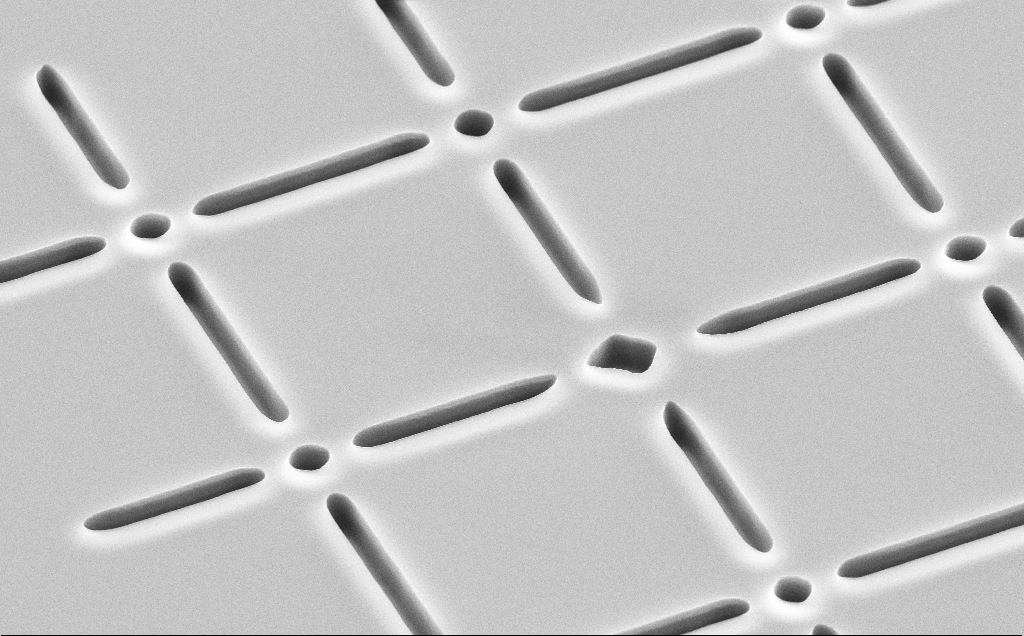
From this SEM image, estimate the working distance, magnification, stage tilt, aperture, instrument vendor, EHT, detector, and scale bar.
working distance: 11 mm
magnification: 6.56 K X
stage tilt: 45°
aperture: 30 µm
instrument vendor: Zeiss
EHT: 10 kV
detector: SE2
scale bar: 10000 nm